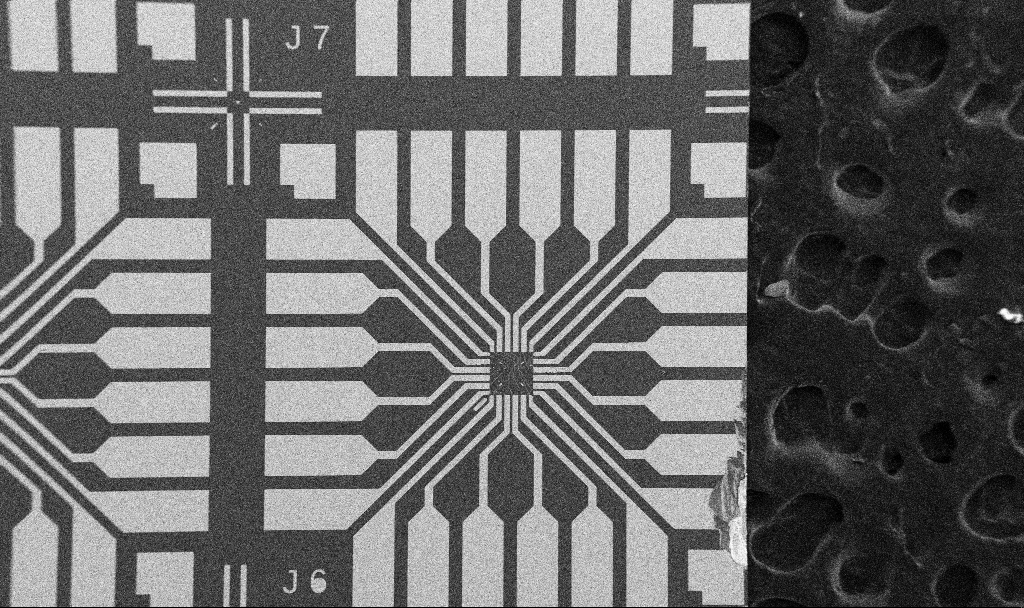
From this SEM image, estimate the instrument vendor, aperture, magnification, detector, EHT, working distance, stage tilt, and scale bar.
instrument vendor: Zeiss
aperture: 30 µm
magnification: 0.1 K X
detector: SE2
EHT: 5 kV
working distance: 10.7 mm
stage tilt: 0°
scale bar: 200000 nm